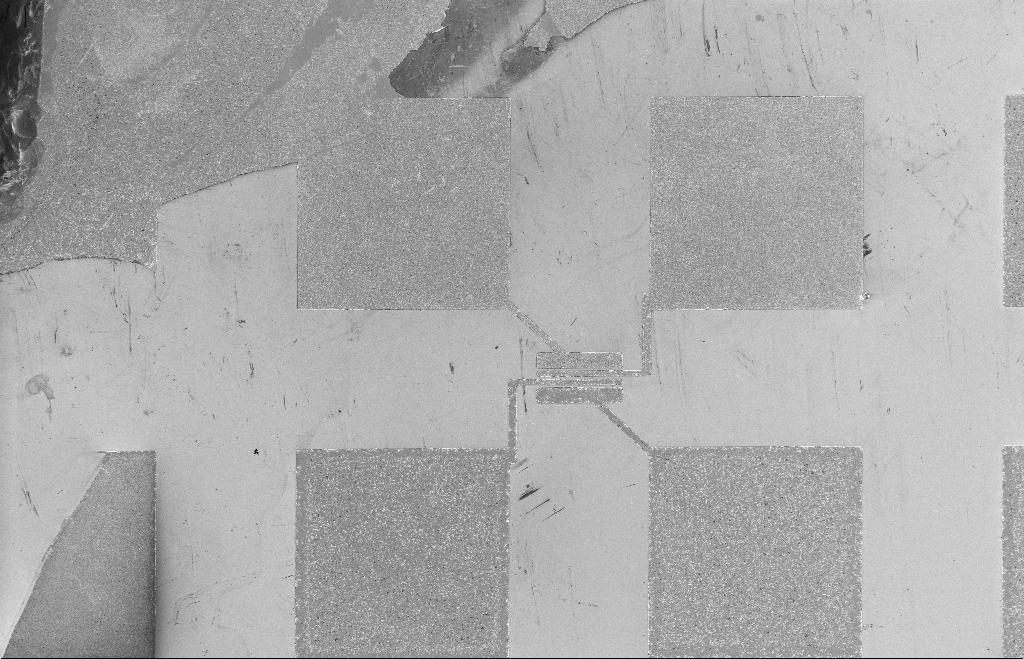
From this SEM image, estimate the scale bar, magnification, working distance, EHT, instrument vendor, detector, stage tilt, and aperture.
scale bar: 20000 nm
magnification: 0.522 K X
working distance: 8 mm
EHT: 5 kV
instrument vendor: Zeiss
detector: InLens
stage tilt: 0°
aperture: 20 µm